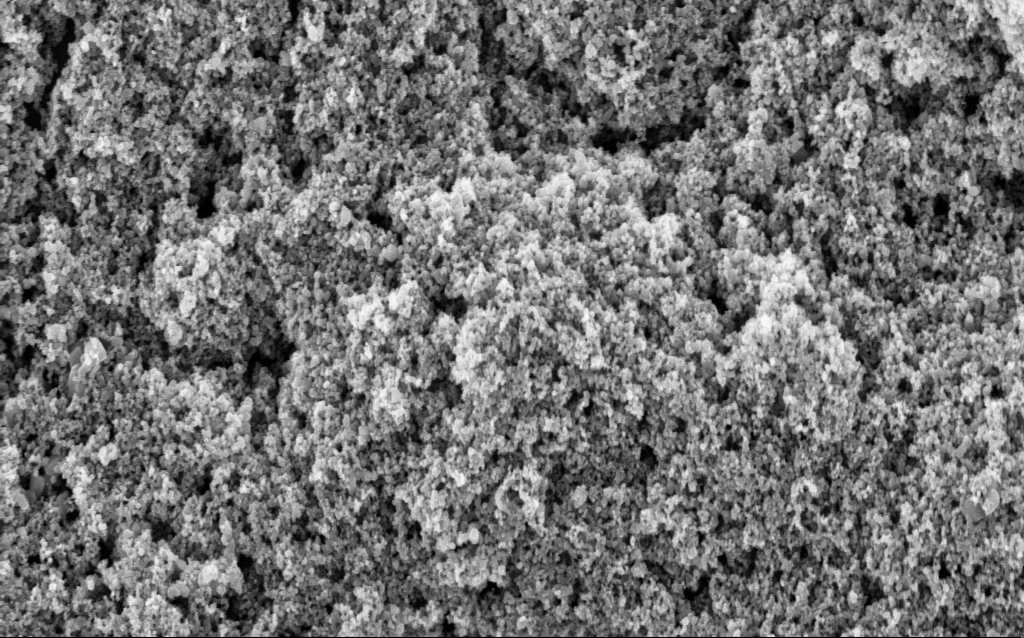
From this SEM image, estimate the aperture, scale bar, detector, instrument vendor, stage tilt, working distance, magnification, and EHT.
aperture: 30 µm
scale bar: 1000 nm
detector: InLens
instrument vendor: Zeiss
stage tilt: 0°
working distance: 9.6 mm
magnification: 46.99 K X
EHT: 5 kV